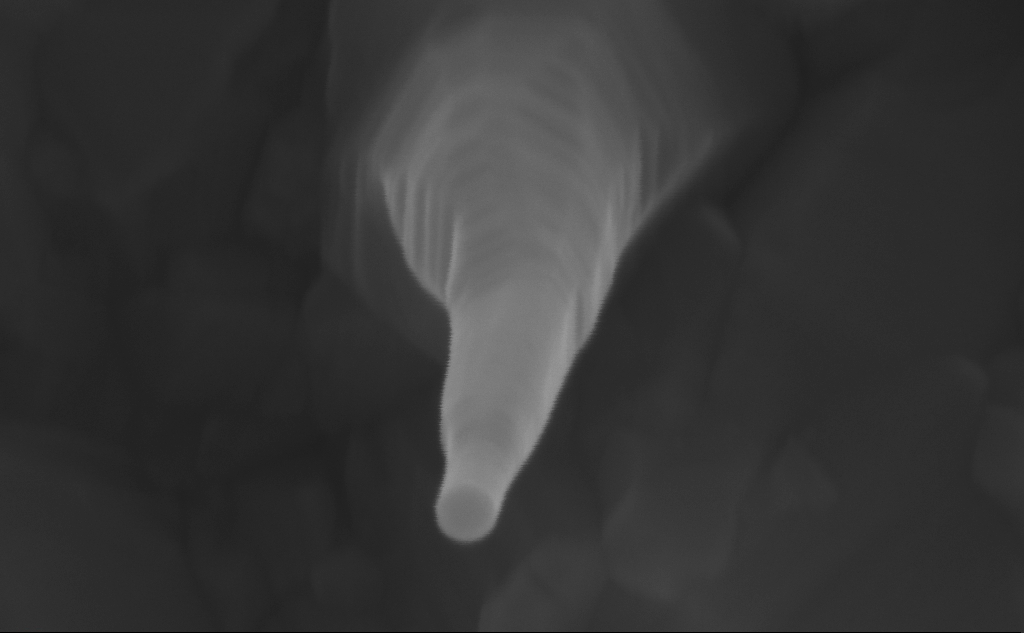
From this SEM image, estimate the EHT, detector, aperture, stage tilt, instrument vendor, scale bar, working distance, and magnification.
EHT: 10 kV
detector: InLens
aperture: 30 µm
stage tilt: -0°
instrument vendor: Zeiss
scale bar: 100 nm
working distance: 7 mm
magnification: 439.9 K X